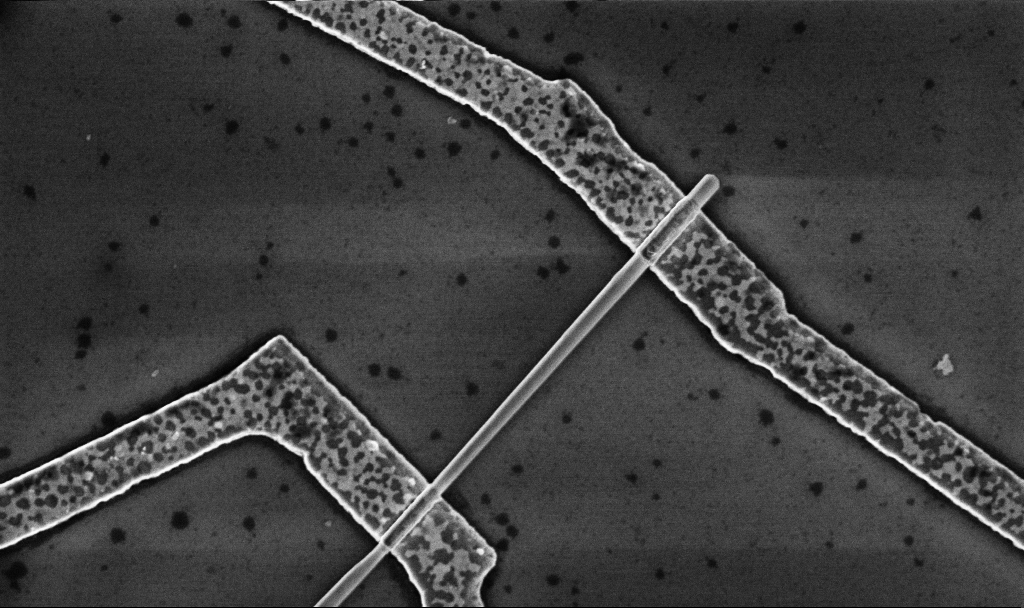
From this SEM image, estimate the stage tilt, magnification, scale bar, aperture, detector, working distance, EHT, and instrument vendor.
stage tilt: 0°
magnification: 30 K X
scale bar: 1000 nm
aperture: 30 µm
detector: InLens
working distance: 8.7 mm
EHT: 5 kV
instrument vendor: Zeiss